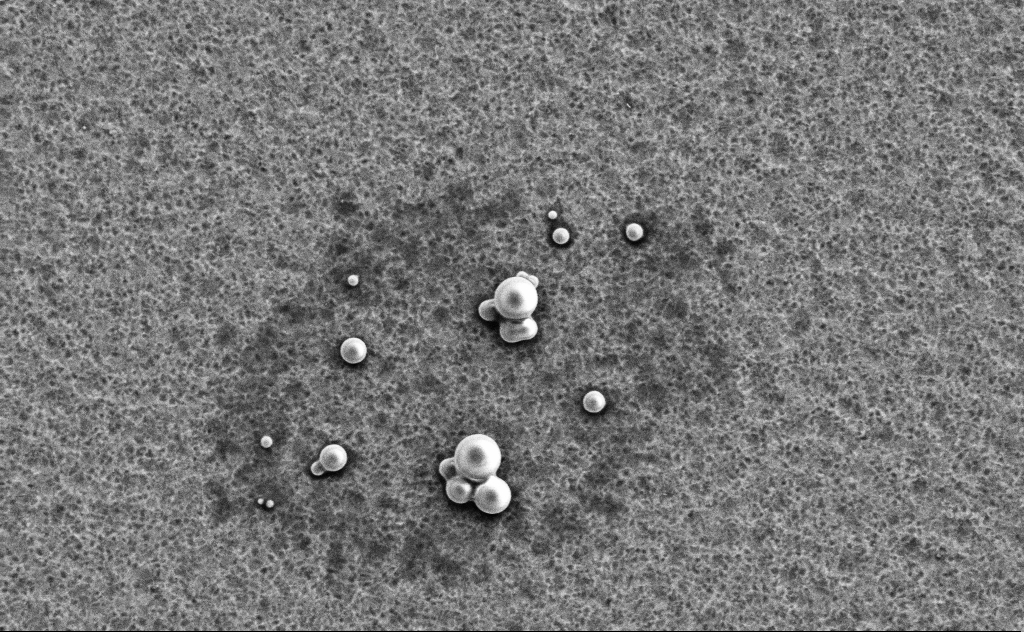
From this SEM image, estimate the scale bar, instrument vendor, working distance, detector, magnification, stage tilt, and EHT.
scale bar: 2000 nm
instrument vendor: Zeiss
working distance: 13 mm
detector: SE2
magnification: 9.79 K X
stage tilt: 0°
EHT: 3 kV